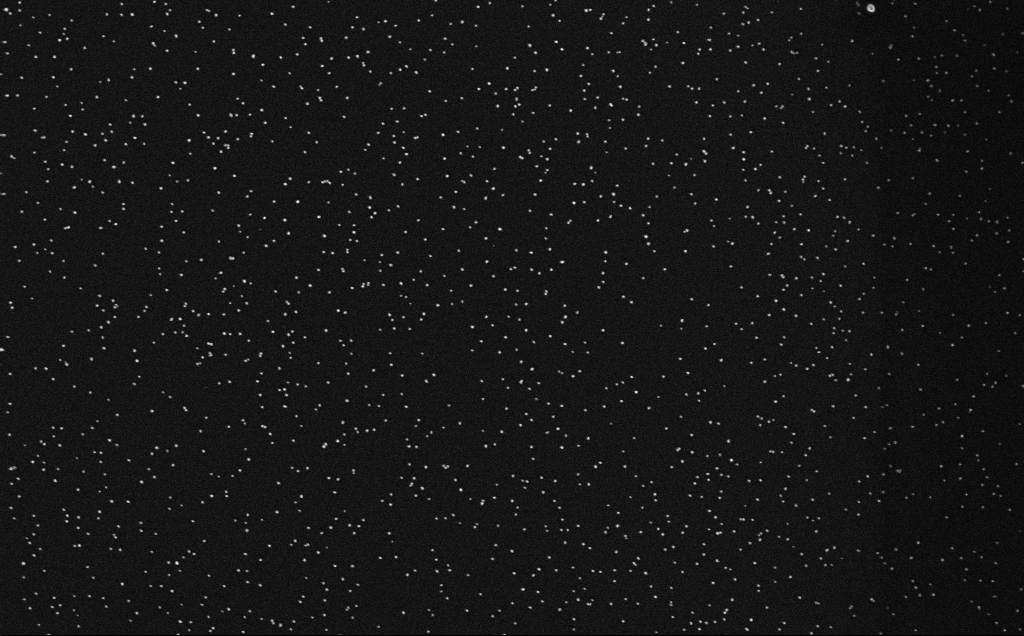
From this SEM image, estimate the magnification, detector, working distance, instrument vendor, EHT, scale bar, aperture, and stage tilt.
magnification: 100 K X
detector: InLens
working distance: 4 mm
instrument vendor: Zeiss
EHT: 10 kV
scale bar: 200 nm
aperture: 30 µm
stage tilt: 0°